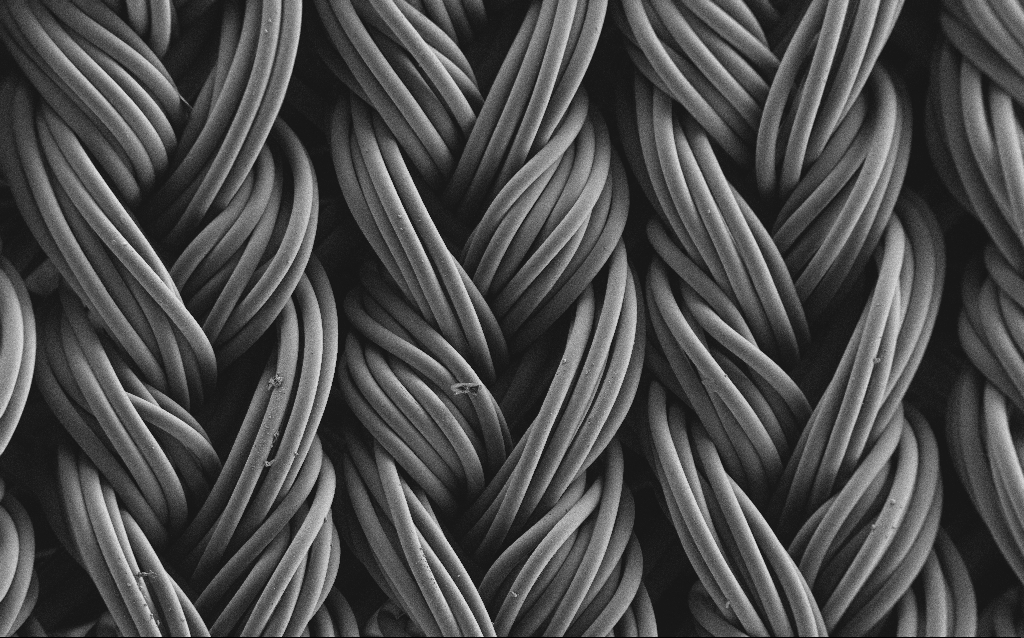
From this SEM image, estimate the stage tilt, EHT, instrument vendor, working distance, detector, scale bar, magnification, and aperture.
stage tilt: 0°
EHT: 1 kV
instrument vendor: Zeiss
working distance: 4 mm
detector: SE2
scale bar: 100000 nm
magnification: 0.282 K X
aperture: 30 µm